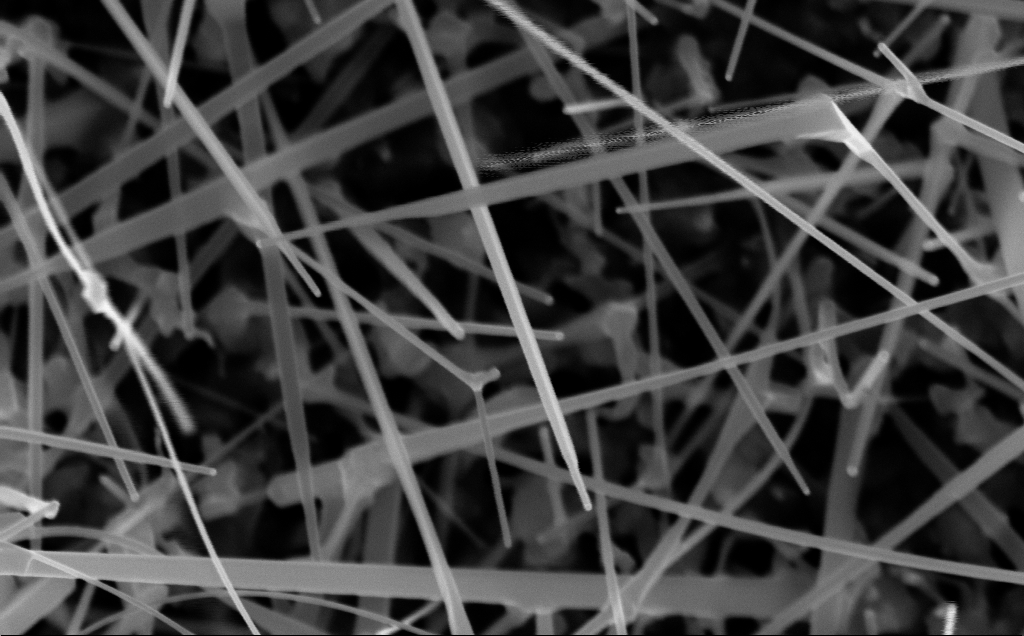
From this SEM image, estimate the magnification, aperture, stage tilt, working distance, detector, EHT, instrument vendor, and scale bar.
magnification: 100 K X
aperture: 30 µm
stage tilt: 0°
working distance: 7 mm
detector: InLens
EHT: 10 kV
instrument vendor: Zeiss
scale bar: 200 nm